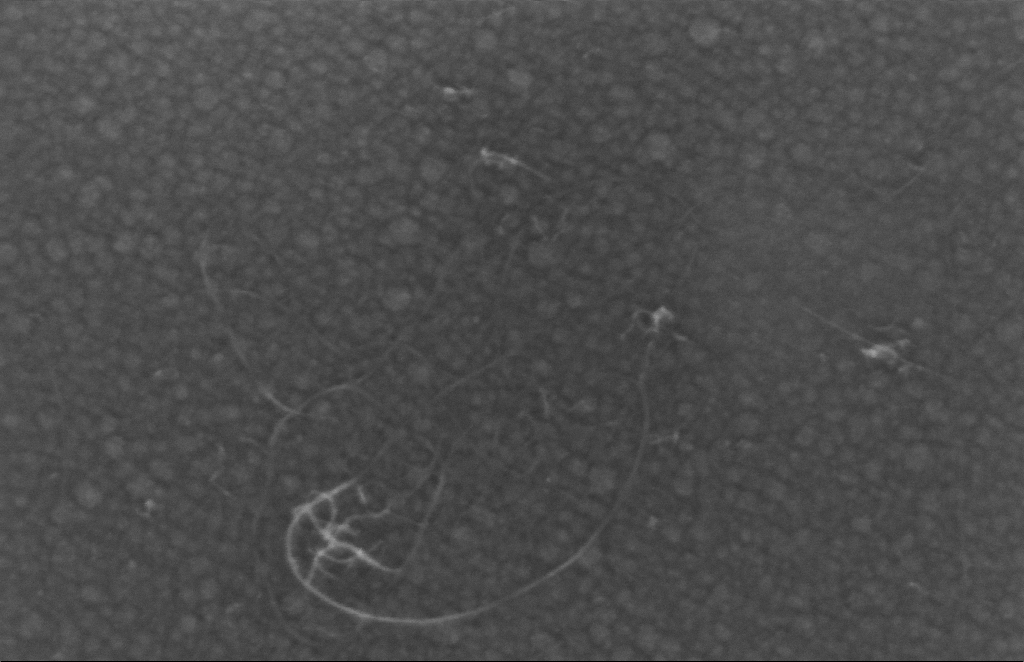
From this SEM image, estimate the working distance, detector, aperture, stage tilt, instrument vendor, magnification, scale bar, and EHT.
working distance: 4 mm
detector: InLens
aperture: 30 µm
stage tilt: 0°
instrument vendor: Zeiss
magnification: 449.49 K X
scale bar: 100 nm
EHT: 5 kV